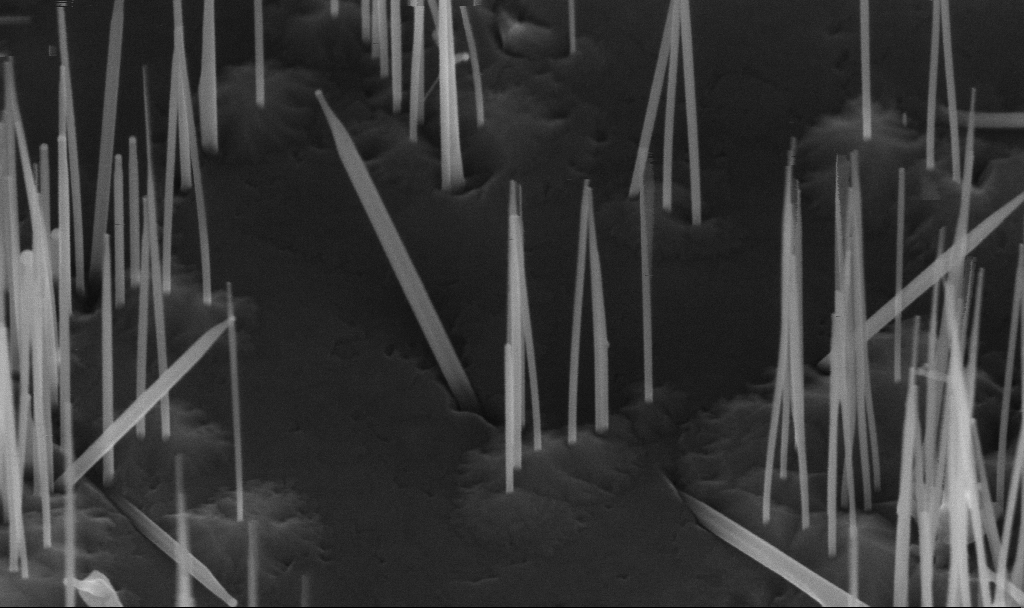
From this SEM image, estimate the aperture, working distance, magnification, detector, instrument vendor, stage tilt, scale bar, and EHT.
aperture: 30 µm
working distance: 5.6 mm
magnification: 127.45 K X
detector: InLens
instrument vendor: Zeiss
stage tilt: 45°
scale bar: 200 nm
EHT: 10 kV